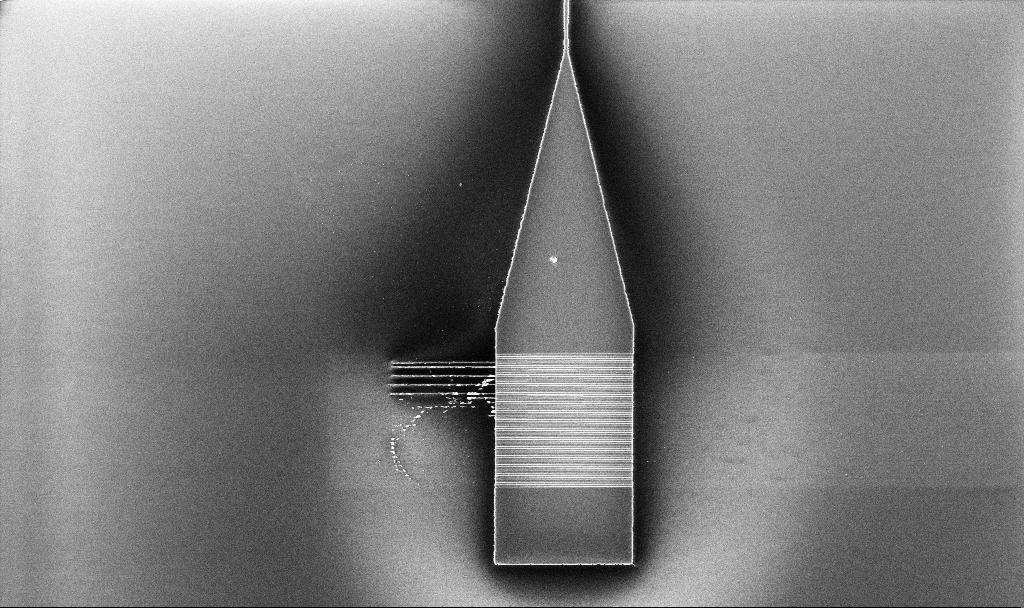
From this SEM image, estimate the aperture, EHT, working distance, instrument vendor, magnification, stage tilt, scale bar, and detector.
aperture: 30 µm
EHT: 5 kV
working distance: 10.1 mm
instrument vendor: Zeiss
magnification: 2.55 K X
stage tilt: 0°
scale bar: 20000 nm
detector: InLens